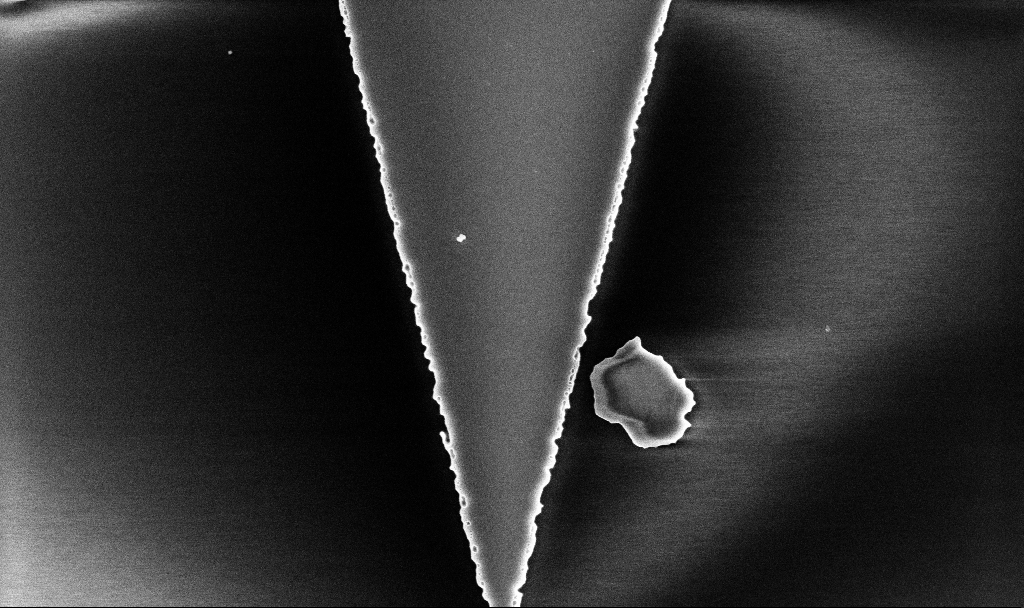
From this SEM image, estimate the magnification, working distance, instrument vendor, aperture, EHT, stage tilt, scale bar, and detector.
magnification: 16.84 K X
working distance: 10.1 mm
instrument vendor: Zeiss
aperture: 30 µm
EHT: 5 kV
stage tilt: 0°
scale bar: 2000 nm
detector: InLens